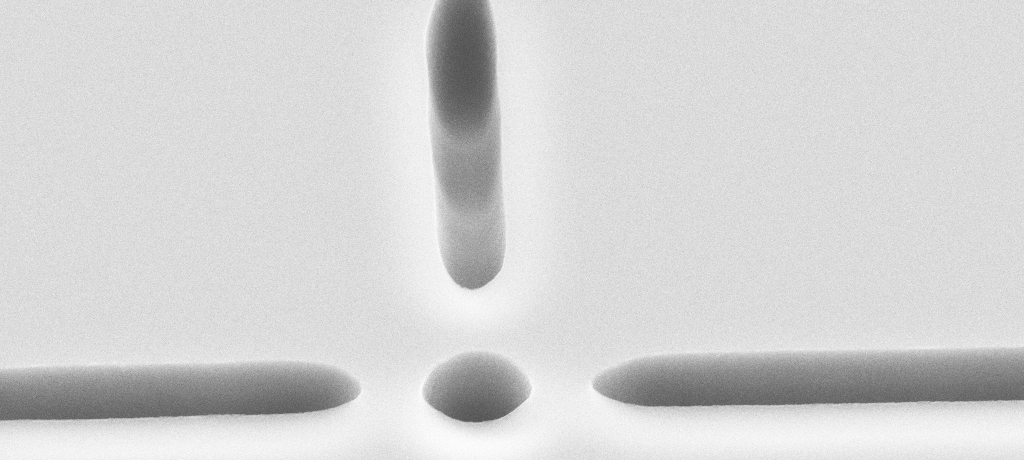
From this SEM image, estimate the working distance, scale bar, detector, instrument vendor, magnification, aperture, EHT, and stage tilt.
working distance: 8 mm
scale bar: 2000 nm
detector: SE2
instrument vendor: Zeiss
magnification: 16.68 K X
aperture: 30 µm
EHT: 10 kV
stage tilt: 45°